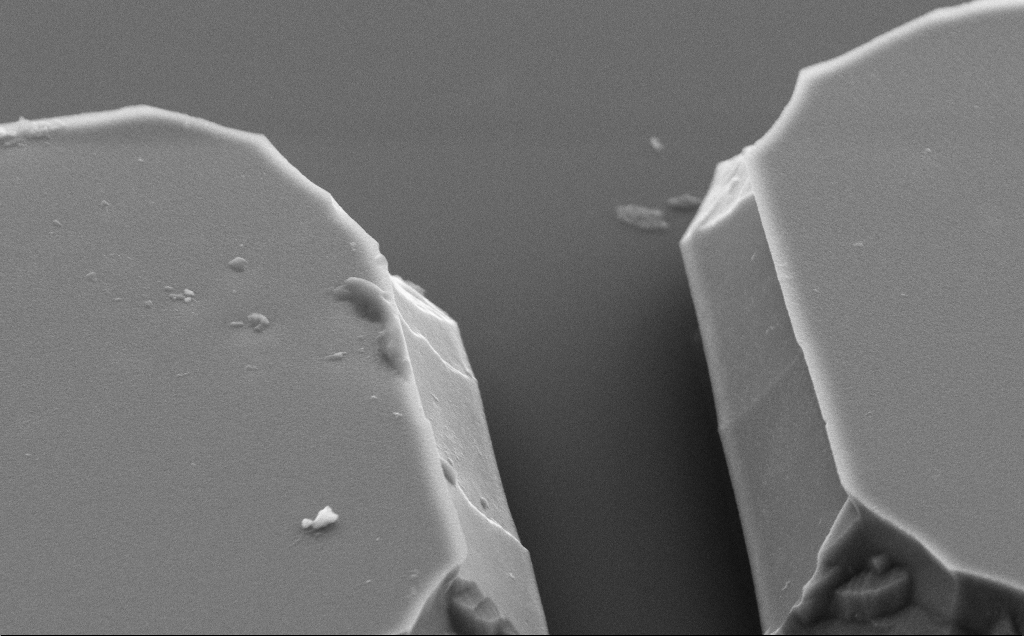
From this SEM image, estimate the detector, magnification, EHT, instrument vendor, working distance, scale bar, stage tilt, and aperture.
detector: SE2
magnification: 22.77 K X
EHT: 5 kV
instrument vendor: Zeiss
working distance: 10 mm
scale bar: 2000 nm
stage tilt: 50°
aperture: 30 µm